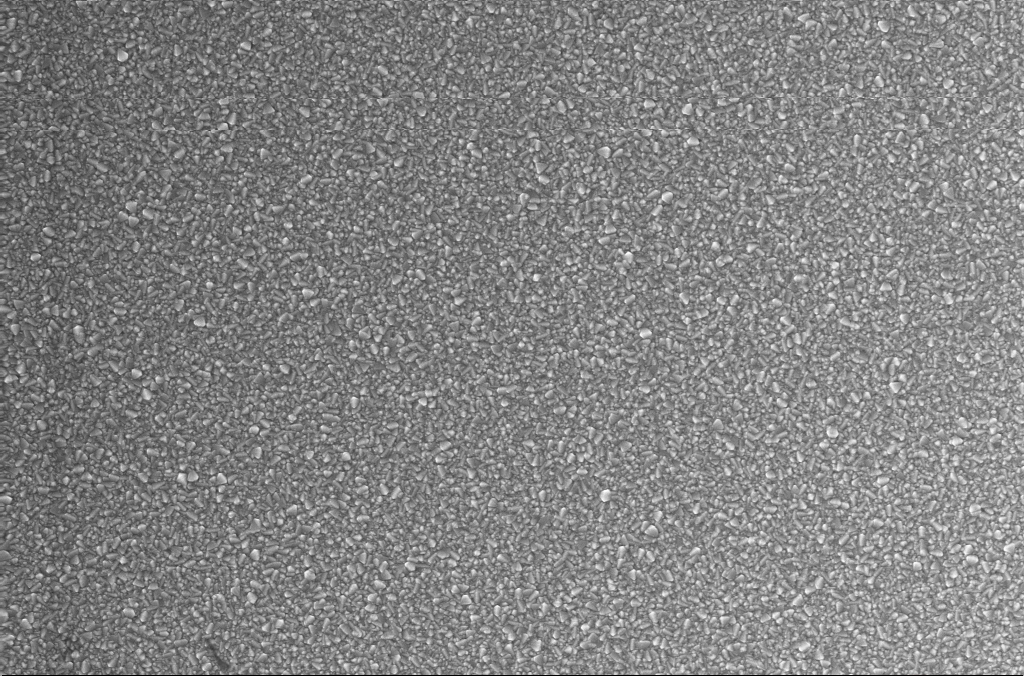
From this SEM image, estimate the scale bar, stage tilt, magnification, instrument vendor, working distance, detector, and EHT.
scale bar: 2000 nm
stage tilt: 0°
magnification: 10 K X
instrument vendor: Zeiss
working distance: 1.9 mm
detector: InLens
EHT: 10 kV